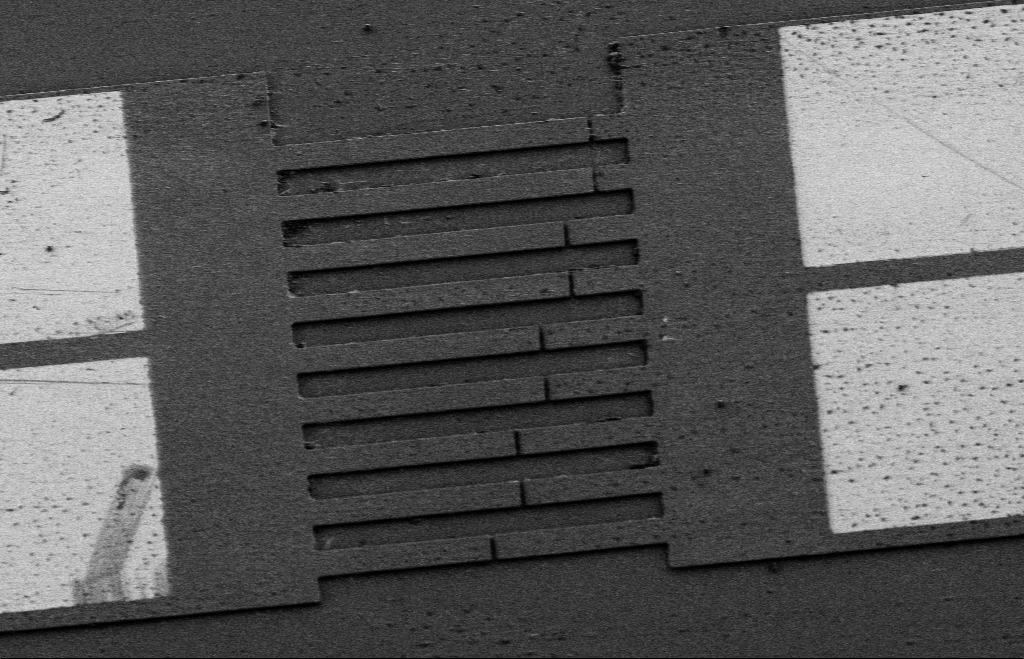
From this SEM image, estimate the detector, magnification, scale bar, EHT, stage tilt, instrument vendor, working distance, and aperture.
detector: SE2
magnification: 0.516 K X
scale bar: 20000 nm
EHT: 5 kV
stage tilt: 23.1°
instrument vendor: Zeiss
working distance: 9 mm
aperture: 30 µm